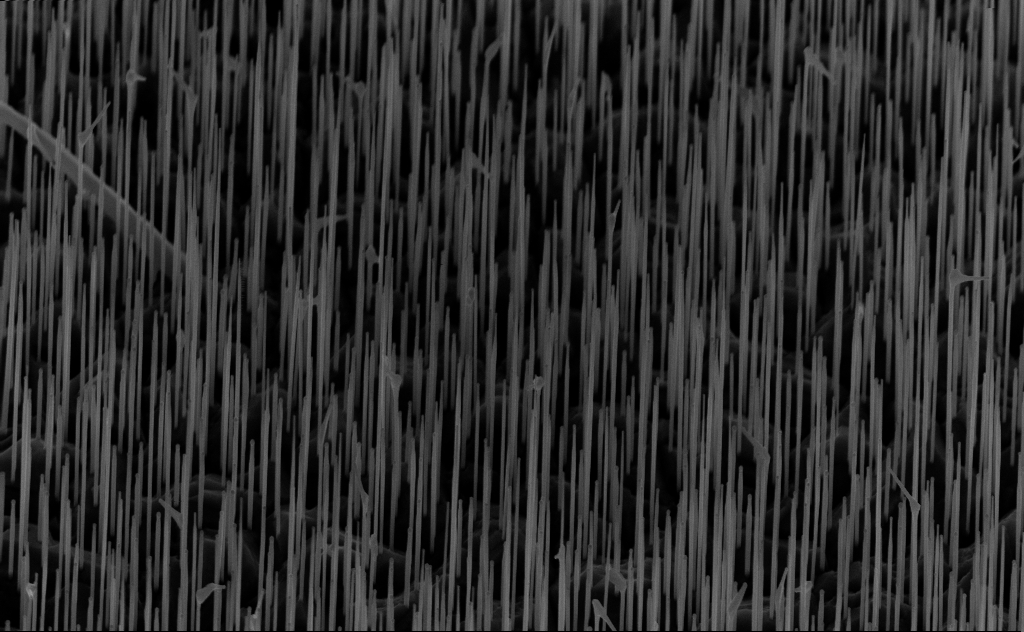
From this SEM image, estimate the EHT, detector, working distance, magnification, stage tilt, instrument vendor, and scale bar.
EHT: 10 kV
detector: InLens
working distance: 6 mm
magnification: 20 K X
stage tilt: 45°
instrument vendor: Zeiss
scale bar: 2000 nm